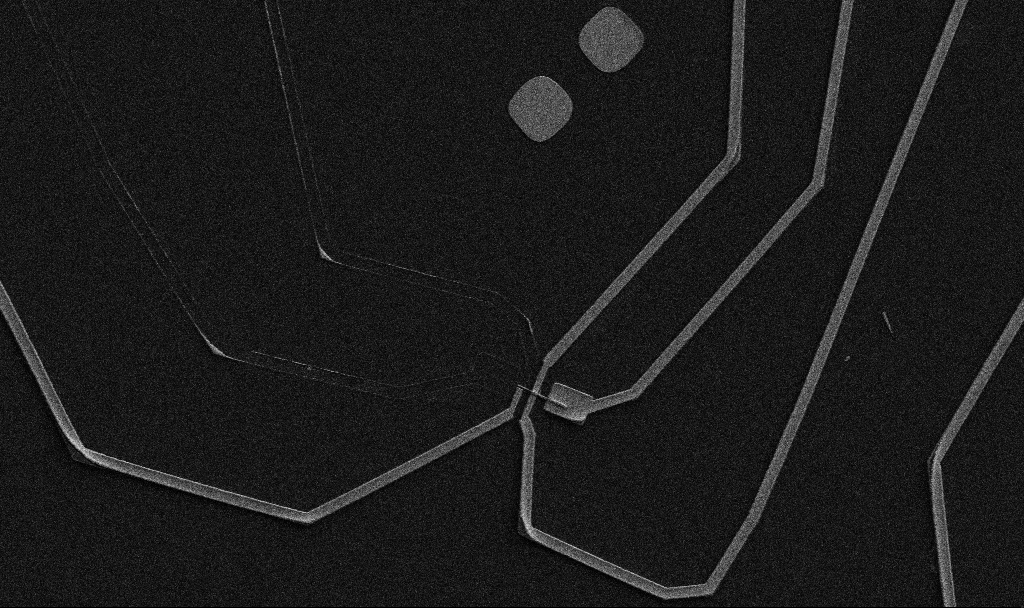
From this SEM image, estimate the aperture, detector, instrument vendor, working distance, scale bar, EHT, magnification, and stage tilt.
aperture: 30 µm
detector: SE2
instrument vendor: Zeiss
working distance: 10.7 mm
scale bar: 10000 nm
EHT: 5 kV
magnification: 5 K X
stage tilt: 0°